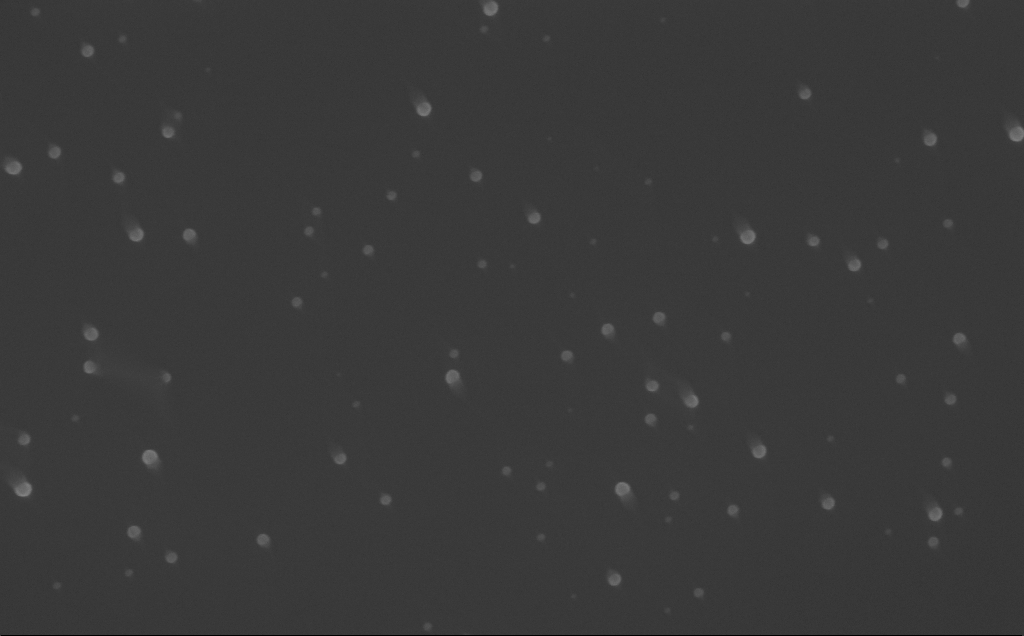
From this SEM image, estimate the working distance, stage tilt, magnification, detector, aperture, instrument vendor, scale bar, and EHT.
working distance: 6 mm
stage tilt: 0°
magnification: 80 K X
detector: InLens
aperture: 30 µm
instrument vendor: Zeiss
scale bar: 200 nm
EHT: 10 kV